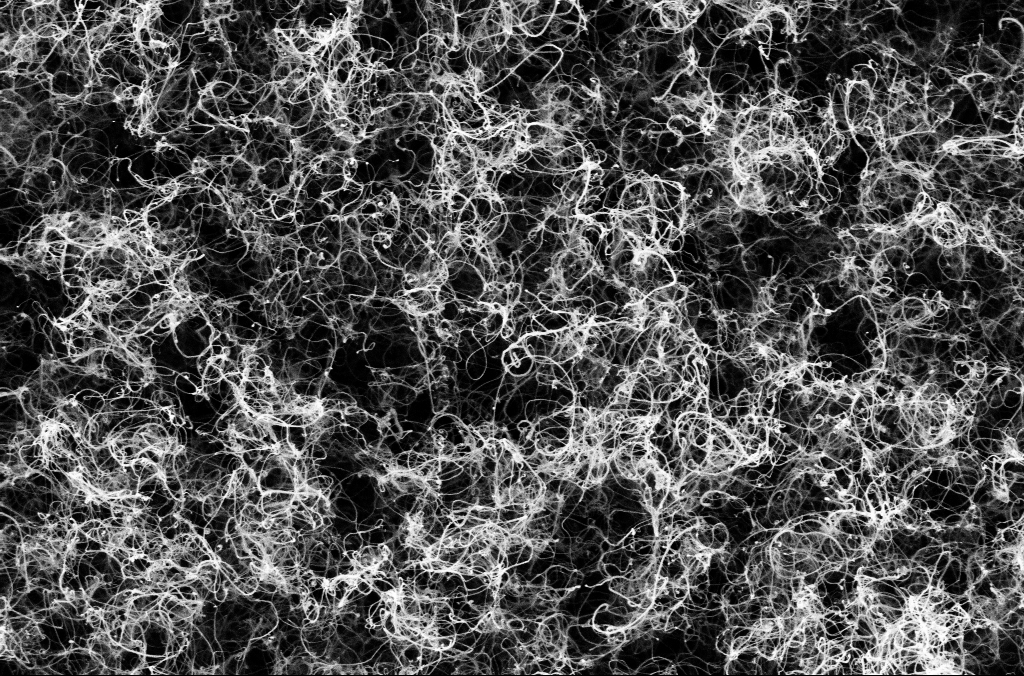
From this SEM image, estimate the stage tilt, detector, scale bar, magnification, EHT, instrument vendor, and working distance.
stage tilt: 0°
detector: SE2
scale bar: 1000 nm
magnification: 25 K X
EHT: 1.8 kV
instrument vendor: Zeiss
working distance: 5.3 mm